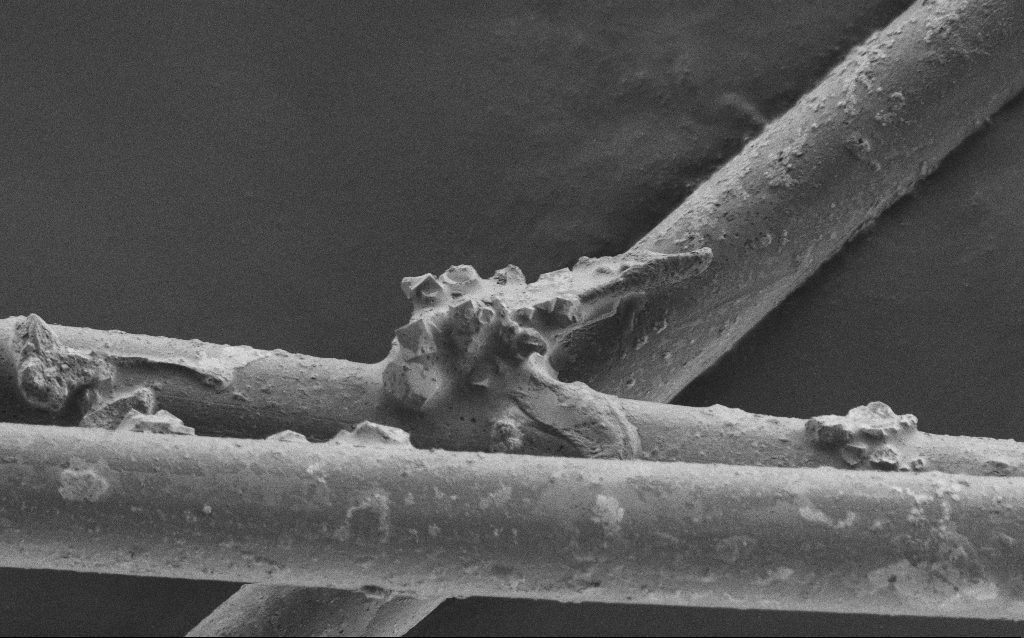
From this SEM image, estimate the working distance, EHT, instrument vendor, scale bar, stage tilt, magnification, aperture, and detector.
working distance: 5 mm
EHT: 1 kV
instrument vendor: Zeiss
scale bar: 10000 nm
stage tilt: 0°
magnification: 2.28 K X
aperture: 30 µm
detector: SE2